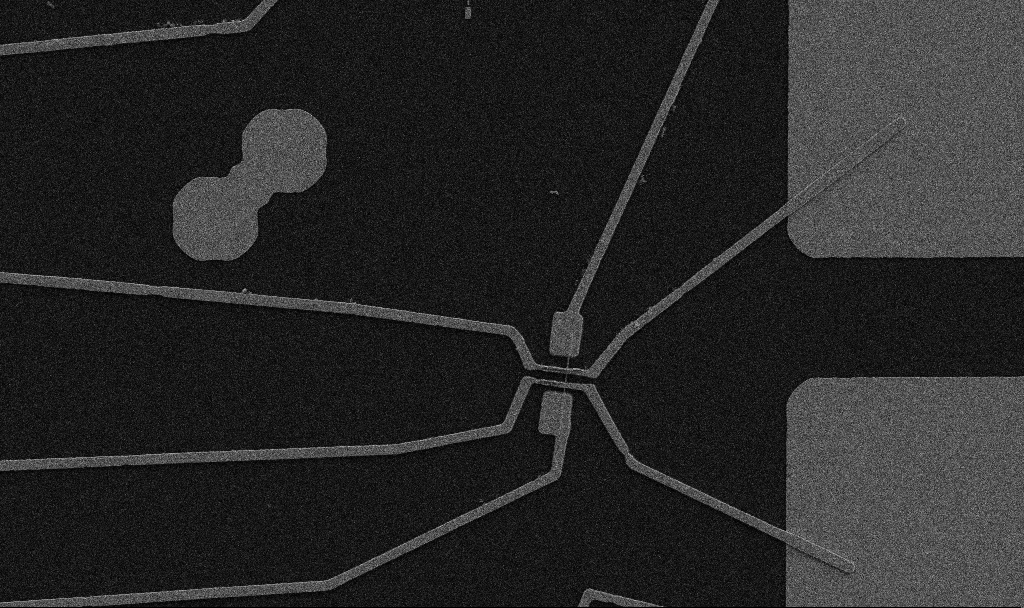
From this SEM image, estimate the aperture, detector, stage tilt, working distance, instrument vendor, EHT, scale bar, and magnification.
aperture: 30 µm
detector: SE2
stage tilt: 0°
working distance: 10.7 mm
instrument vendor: Zeiss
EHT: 5 kV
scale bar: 10000 nm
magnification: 5 K X